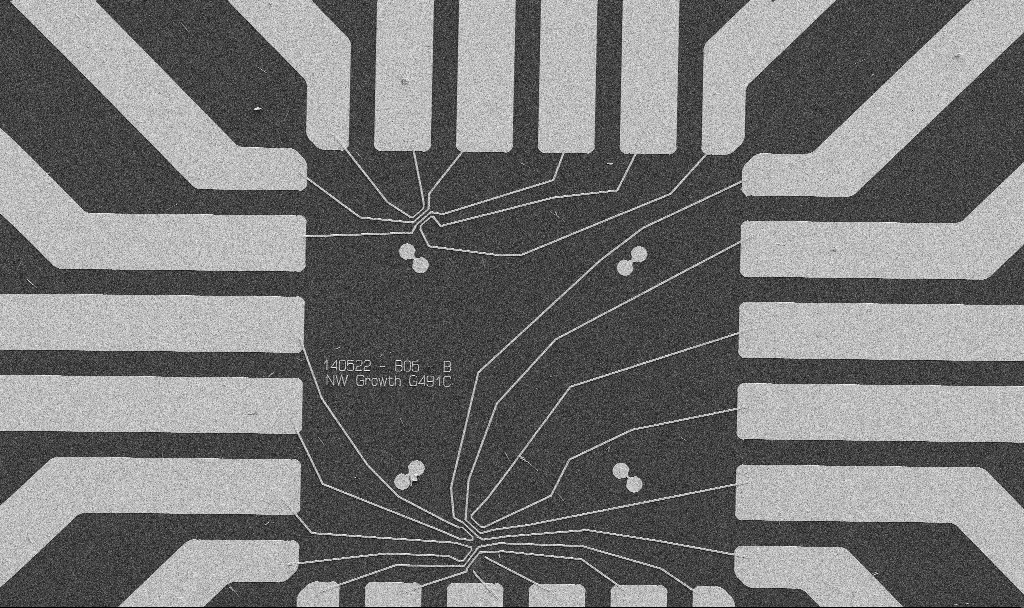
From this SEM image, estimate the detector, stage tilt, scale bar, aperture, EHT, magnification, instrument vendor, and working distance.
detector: SE2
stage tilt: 0°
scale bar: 20000 nm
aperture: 30 µm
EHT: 5 kV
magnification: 1 K X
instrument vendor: Zeiss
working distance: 10.7 mm